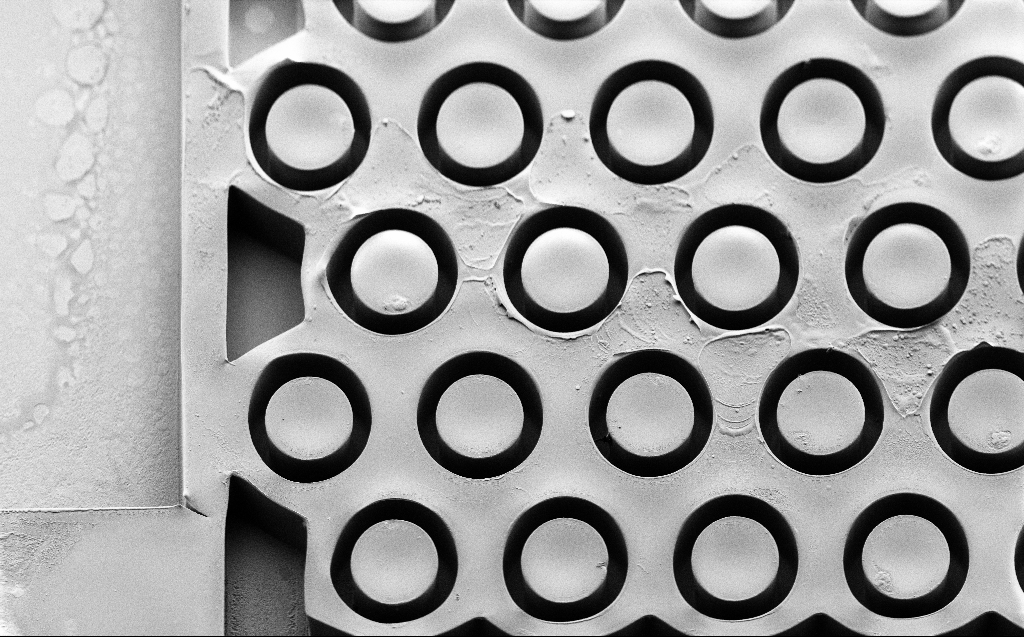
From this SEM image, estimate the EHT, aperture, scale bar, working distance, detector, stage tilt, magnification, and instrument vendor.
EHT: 2 kV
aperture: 30 µm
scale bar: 20000 nm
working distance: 7 mm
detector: SE2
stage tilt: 45°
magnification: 0.785 K X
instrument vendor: Zeiss